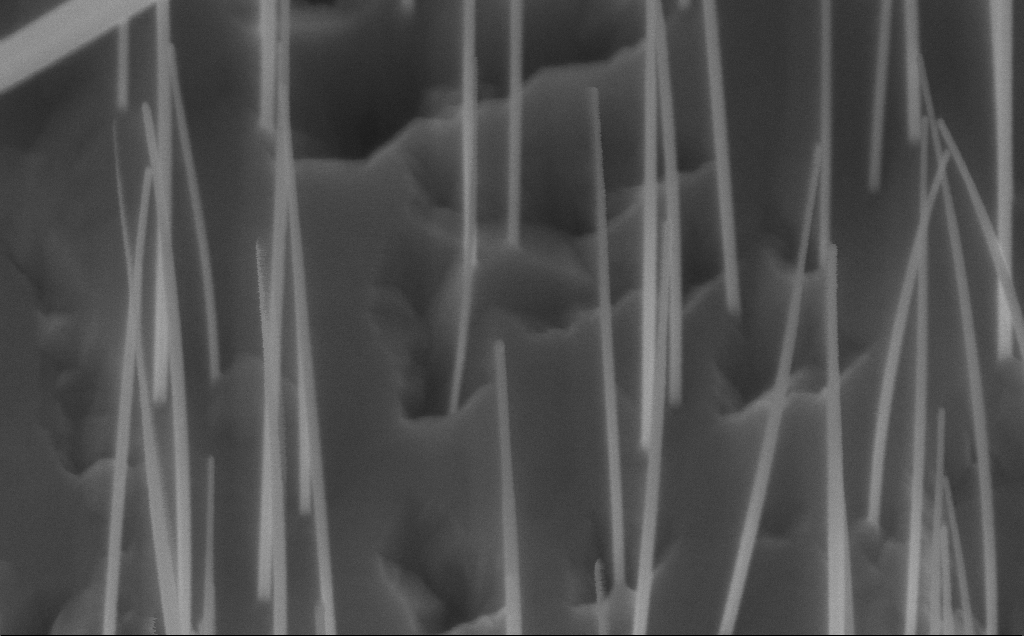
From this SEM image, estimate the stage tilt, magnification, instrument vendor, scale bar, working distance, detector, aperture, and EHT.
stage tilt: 45°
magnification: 150 K X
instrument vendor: Zeiss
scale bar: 200 nm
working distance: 7 mm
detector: InLens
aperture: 30 µm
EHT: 10 kV